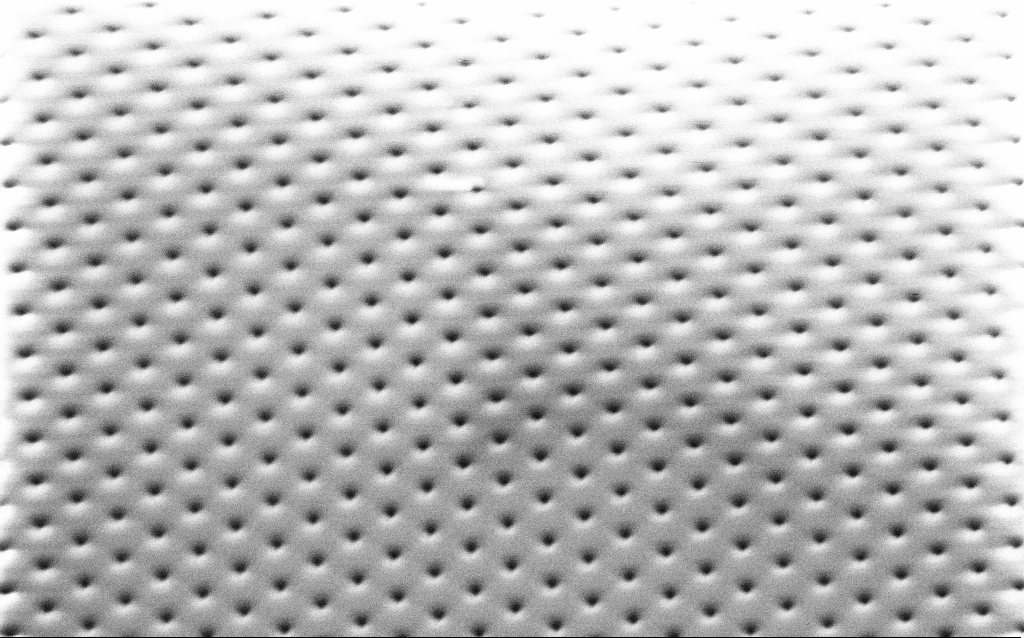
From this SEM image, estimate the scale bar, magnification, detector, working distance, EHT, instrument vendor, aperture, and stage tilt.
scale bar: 2000 nm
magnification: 13.4 K X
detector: SE2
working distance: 9.6 mm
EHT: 1.5 kV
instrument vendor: Zeiss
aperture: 30 µm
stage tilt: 45°